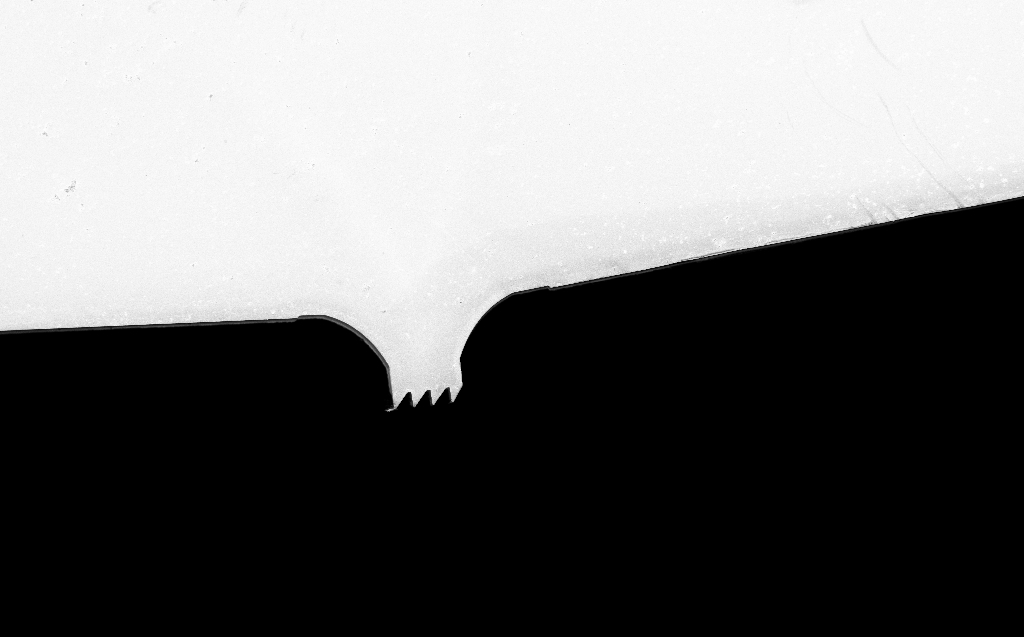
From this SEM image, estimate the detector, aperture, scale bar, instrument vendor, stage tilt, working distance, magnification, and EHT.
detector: InLens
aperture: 30 µm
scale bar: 100000 nm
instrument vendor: Zeiss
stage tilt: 0°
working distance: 5 mm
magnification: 0.63 K X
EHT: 3 kV